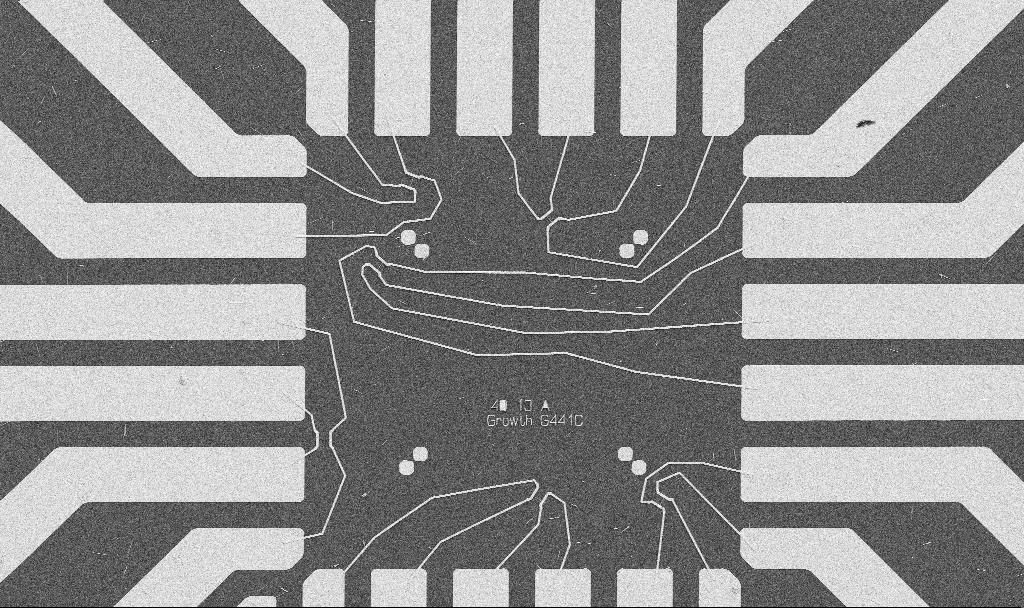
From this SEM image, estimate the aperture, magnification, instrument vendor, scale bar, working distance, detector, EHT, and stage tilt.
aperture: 30 µm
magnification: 1 K X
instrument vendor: Zeiss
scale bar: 20000 nm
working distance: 10.7 mm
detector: SE2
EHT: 5 kV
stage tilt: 0°